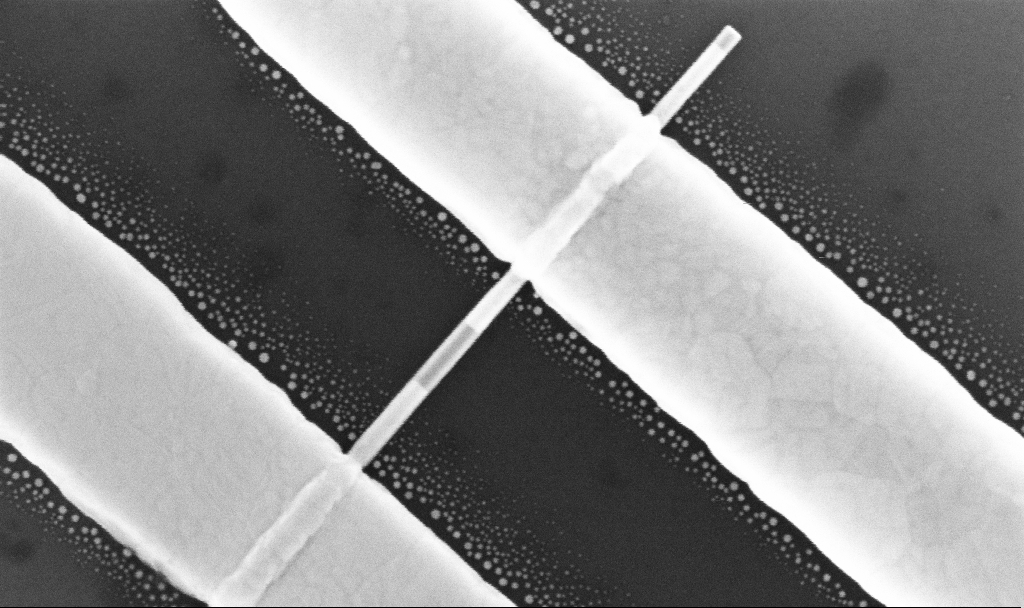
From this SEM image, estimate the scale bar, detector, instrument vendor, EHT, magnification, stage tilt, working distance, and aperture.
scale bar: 200 nm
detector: InLens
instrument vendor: Zeiss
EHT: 10 kV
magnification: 188.45 K X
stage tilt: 0°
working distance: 7 mm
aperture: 30 µm